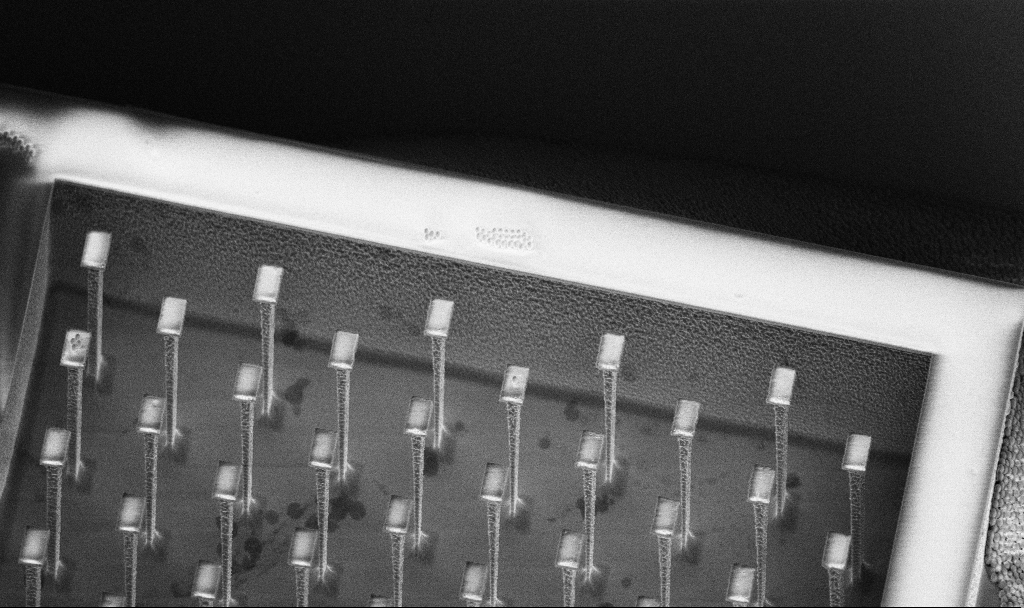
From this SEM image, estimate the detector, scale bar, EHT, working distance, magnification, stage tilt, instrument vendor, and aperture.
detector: InLens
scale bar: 10000 nm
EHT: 5 kV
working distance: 5.9 mm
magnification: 3.09 K X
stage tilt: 45°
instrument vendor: Zeiss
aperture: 30 µm